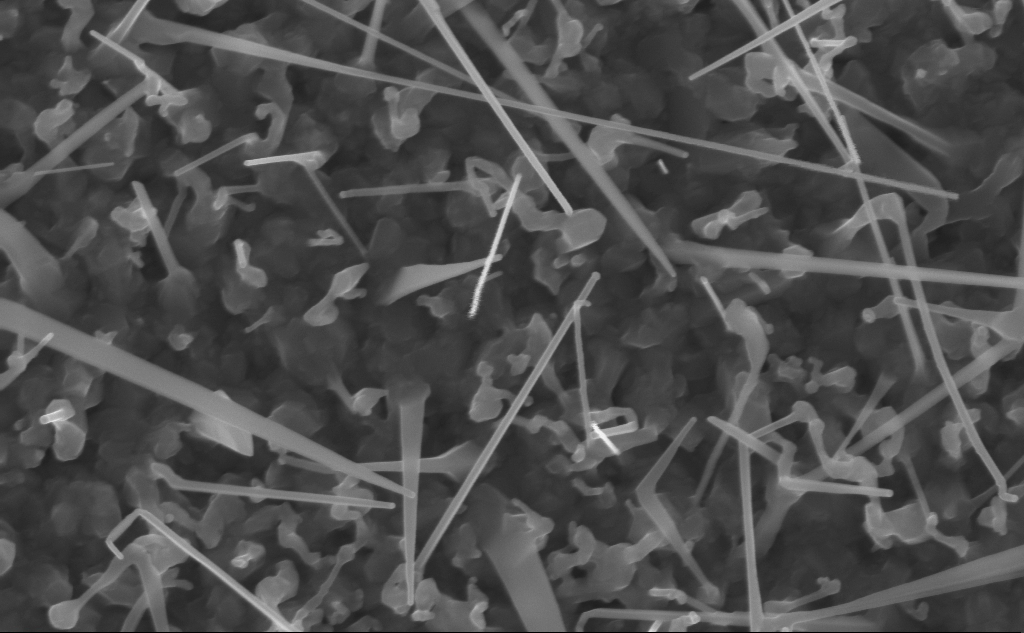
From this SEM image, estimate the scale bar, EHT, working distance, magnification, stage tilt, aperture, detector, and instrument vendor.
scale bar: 200 nm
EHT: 10 kV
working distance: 5 mm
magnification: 80 K X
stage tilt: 0°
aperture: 30 µm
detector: InLens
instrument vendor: Zeiss